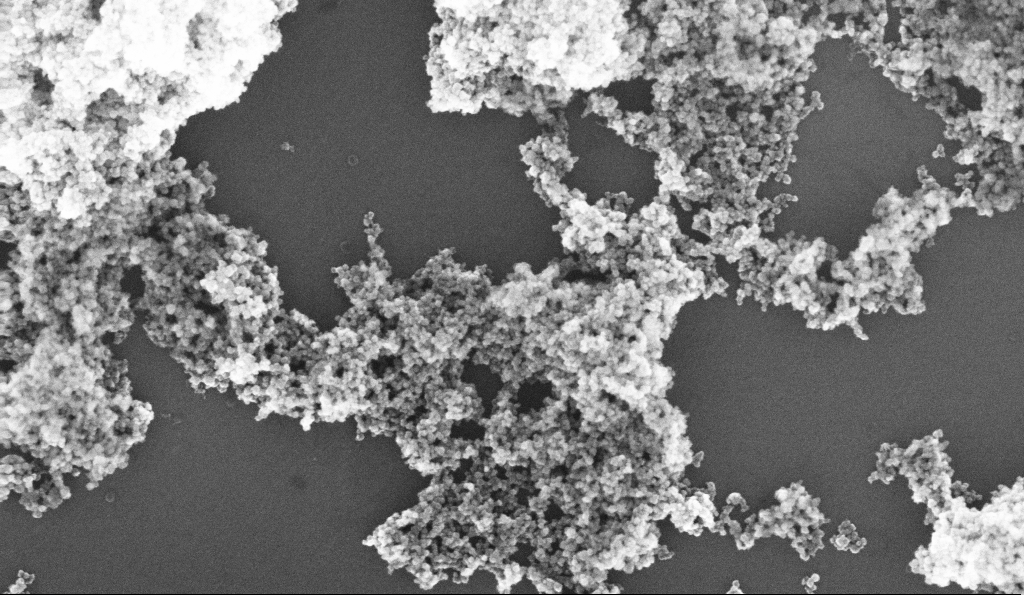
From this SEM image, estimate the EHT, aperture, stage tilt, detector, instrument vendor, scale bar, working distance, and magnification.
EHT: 10 kV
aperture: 30 µm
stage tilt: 0°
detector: InLens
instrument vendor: Zeiss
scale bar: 200 nm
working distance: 5.2 mm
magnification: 131.21 K X